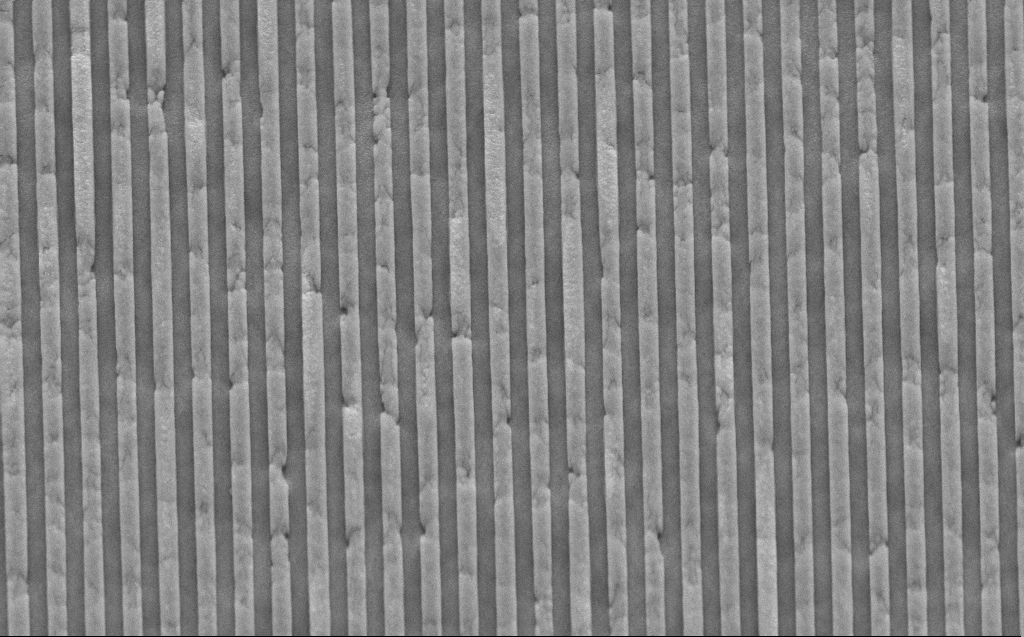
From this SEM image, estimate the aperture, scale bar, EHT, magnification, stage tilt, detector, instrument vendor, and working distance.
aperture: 30 µm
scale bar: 2000 nm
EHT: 10 kV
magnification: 27.75 K X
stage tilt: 45°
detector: SE2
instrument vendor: Zeiss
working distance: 8 mm